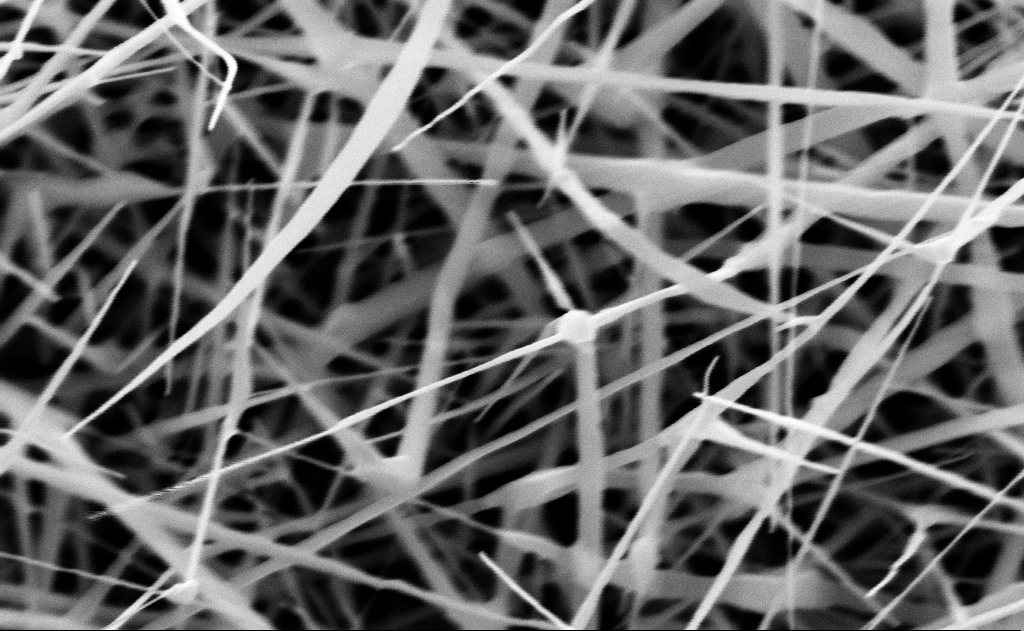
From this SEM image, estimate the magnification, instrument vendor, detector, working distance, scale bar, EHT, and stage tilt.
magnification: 80 K X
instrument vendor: Zeiss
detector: InLens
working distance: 15 mm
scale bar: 200 nm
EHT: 10 kV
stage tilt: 0°